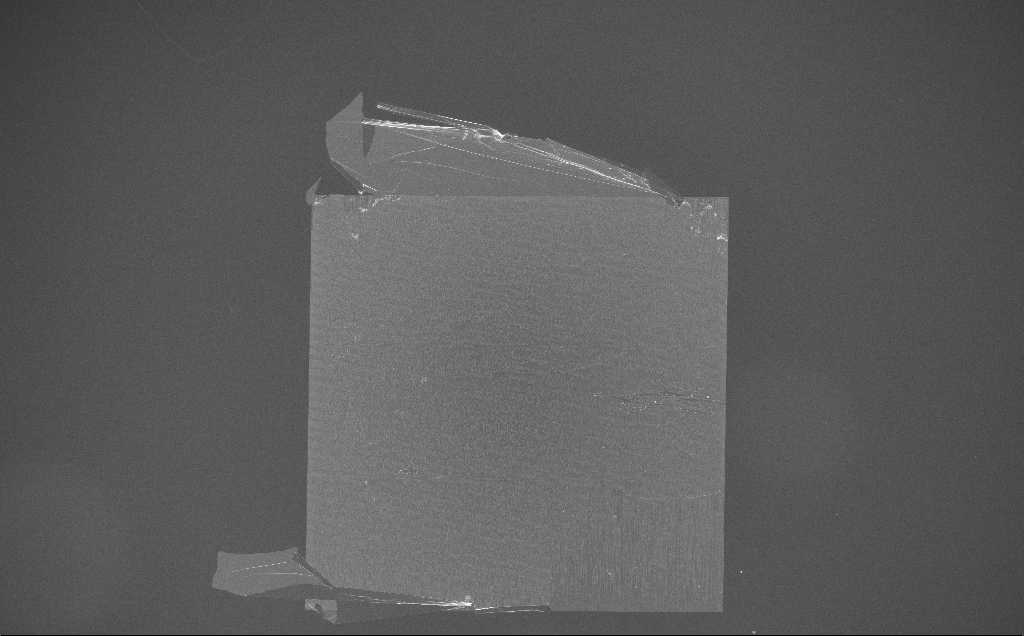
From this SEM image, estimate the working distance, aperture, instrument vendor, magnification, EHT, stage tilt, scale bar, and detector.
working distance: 7 mm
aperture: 30 µm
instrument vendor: Zeiss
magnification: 0.156 K X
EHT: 10 kV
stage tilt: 0°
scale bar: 100000 nm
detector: InLens